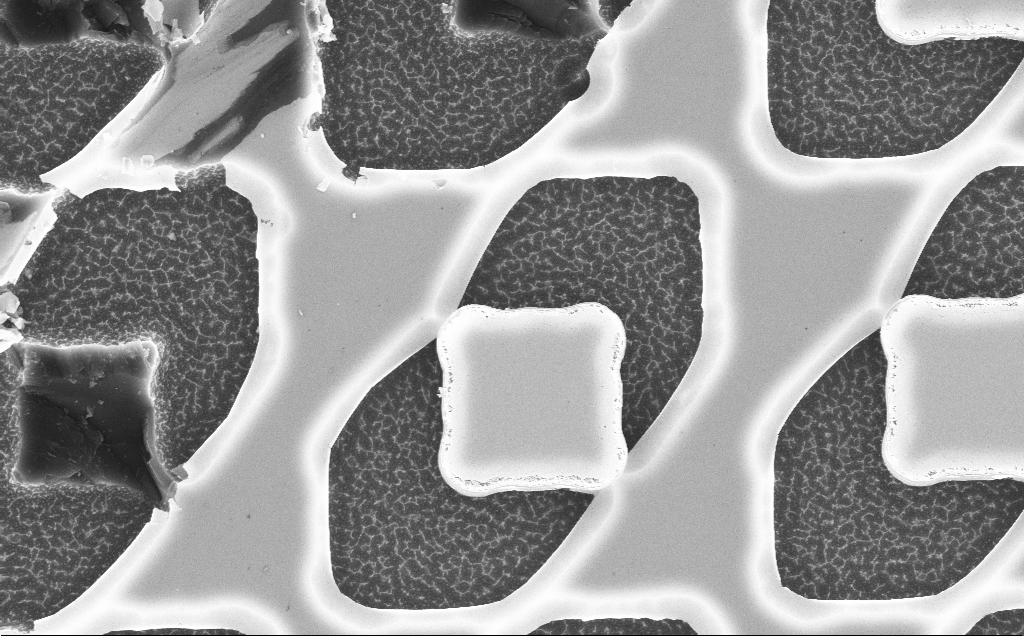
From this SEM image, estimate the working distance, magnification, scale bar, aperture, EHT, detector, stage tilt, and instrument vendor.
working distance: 9 mm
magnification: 8.16 K X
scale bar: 2000 nm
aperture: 30 µm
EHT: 10 kV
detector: InLens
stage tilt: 0°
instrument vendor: Zeiss